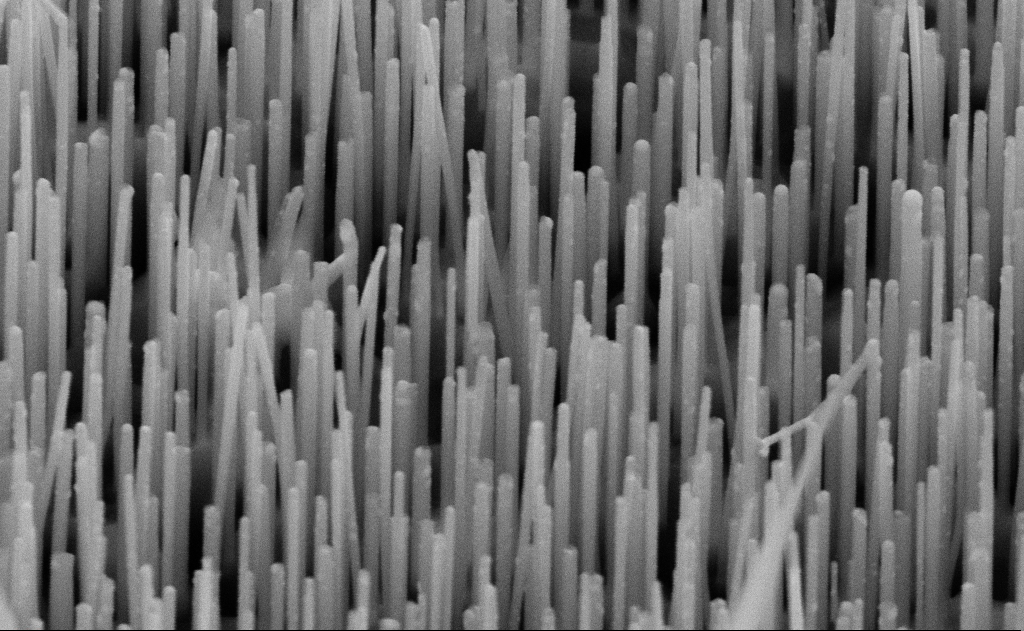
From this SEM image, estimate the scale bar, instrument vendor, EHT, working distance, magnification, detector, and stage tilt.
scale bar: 200 nm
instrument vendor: Zeiss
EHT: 10 kV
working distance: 11 mm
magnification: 100 K X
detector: SE2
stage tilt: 45°